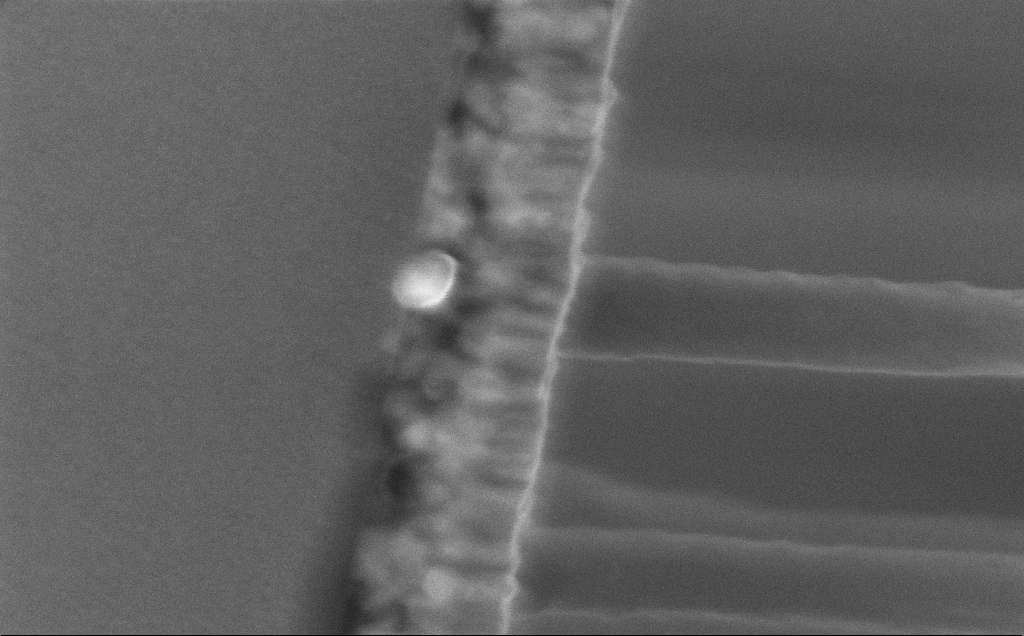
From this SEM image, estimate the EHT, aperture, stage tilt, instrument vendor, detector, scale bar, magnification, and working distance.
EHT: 5 kV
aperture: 30 µm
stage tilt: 0°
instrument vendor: Zeiss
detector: InLens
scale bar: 200 nm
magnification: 243.85 K X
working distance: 12 mm